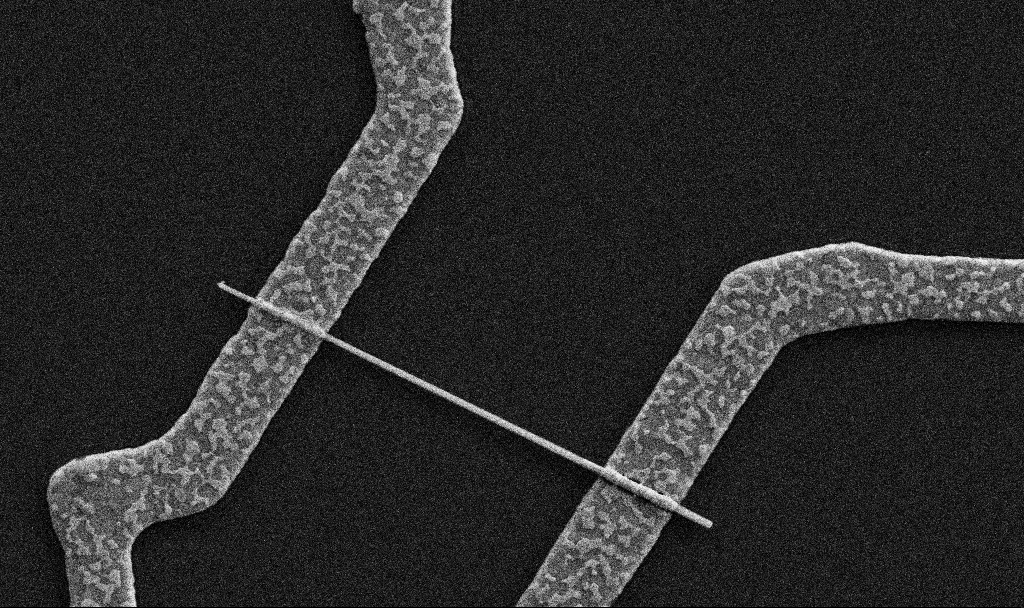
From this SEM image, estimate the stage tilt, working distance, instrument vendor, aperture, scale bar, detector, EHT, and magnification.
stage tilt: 0°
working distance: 6.7 mm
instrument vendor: Zeiss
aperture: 30 µm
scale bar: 2000 nm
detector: SE2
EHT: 5 kV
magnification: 31.89 K X